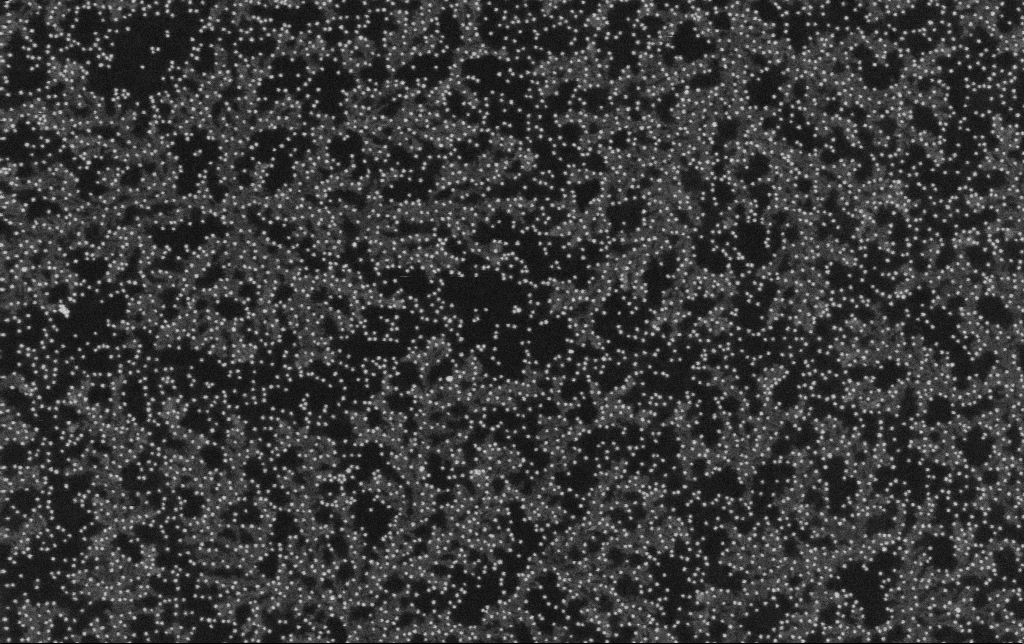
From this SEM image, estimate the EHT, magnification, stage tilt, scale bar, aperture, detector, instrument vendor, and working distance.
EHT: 10 kV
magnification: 100 K X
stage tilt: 0°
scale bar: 200 nm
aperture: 30 µm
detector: SE2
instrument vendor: Zeiss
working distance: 11.3 mm